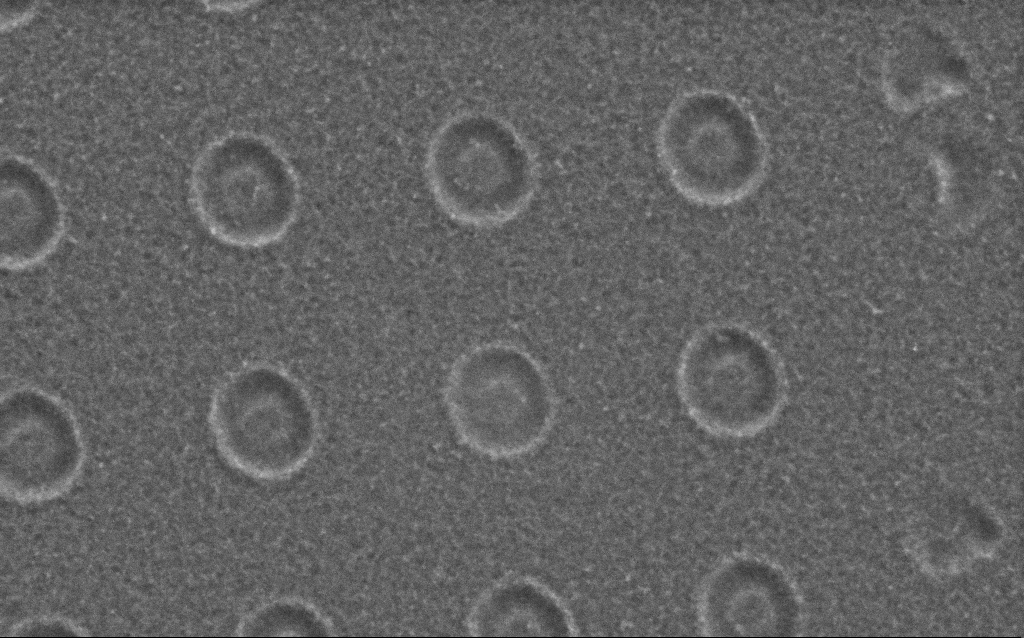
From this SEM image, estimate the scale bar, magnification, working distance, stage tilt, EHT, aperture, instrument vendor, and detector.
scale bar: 200 nm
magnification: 85.69 K X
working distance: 5 mm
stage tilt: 0°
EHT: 1.5 kV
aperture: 30 µm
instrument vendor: Zeiss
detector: SE2